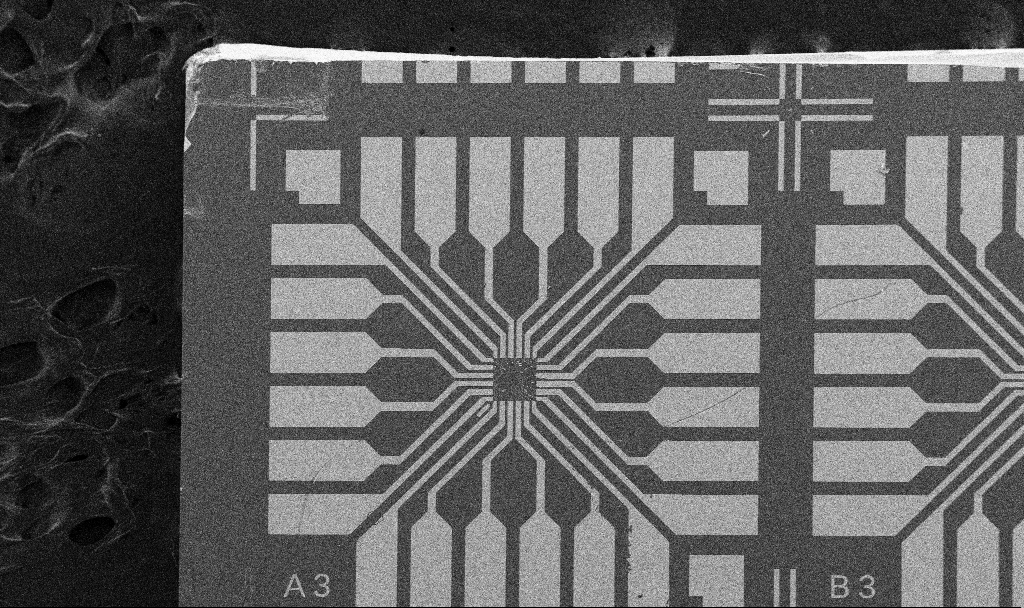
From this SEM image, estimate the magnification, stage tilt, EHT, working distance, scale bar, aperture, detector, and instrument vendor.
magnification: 0.1 K X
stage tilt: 0°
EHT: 10 kV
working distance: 10.7 mm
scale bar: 200000 nm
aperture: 30 µm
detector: SE2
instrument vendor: Zeiss